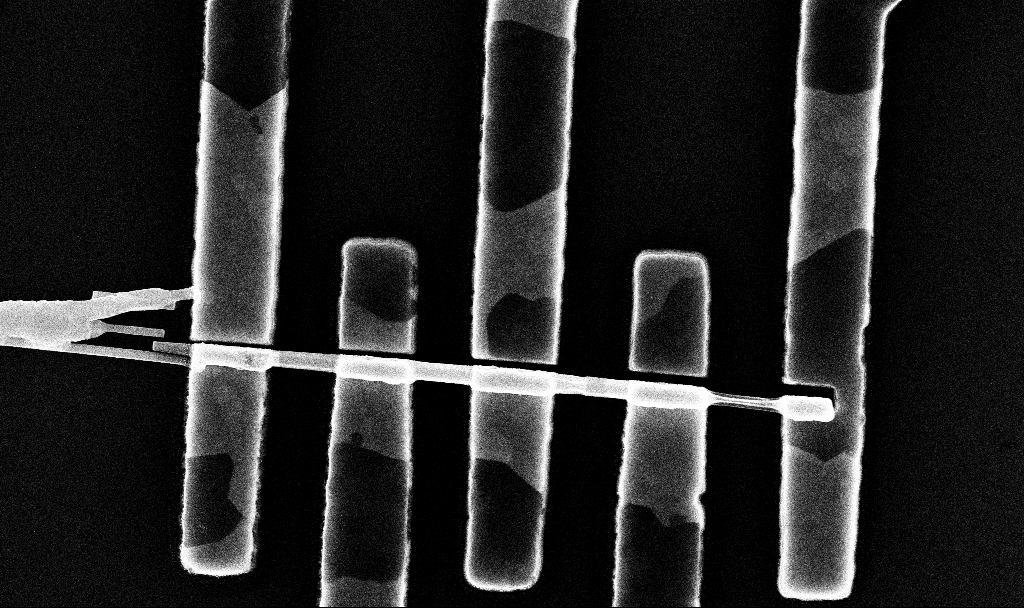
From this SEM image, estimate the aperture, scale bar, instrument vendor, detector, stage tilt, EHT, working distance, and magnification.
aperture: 30 µm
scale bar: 1000 nm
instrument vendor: Zeiss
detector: InLens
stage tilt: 0°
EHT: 10 kV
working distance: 6.8 mm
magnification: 48.94 K X